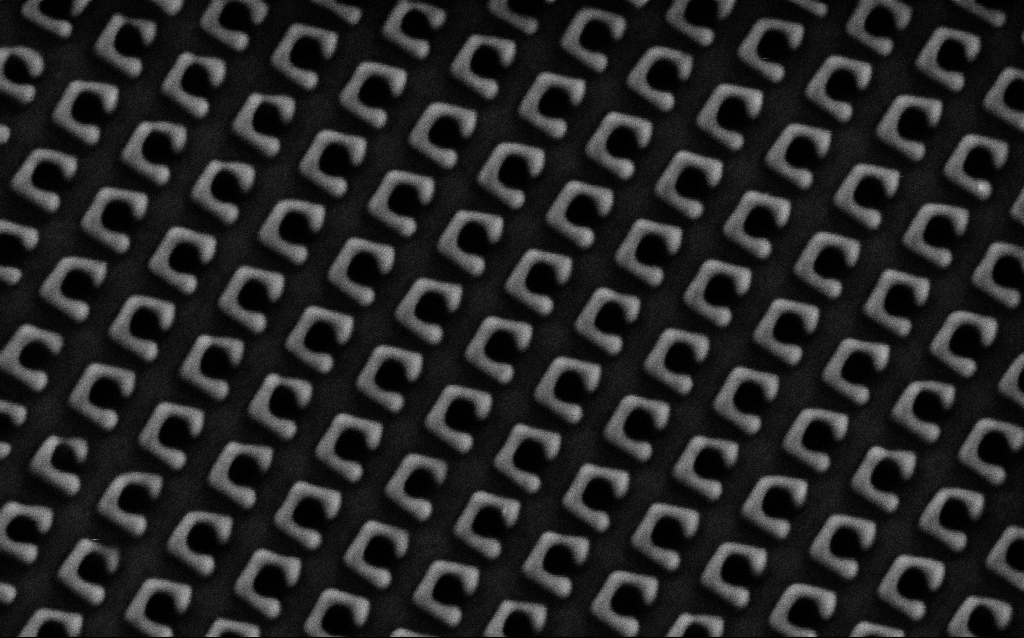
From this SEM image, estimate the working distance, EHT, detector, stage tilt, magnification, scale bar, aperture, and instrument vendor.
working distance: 8 mm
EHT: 1.5 kV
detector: SE2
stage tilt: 0°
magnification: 62.87 K X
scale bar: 1000 nm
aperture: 30 µm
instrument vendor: Zeiss